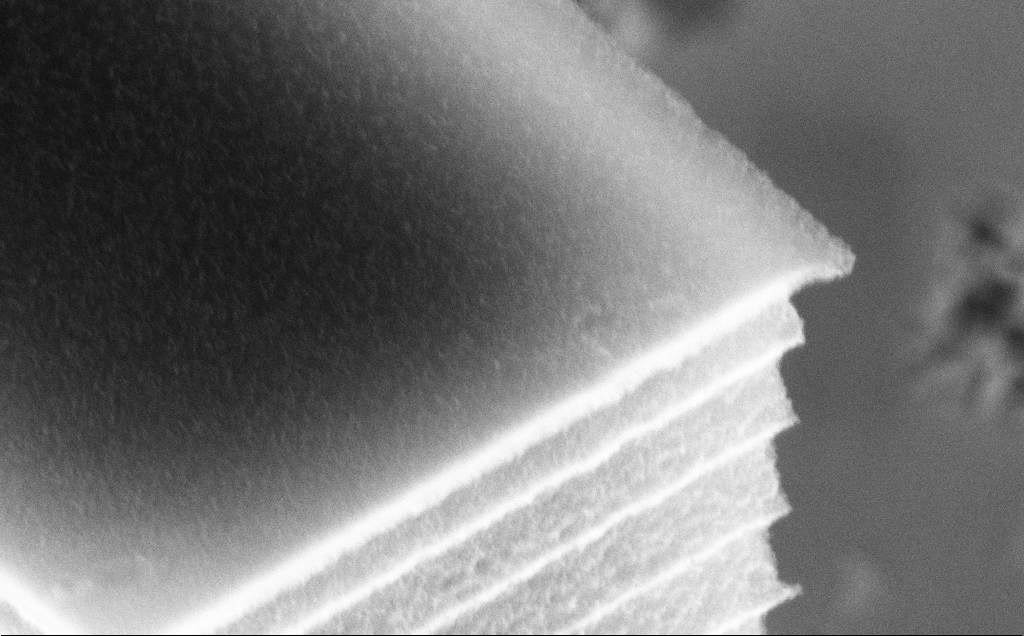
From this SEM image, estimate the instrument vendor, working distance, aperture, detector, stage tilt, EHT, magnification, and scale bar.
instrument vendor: Zeiss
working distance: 7 mm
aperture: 30 µm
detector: InLens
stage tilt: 45°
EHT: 10 kV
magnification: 119.93 K X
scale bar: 200 nm